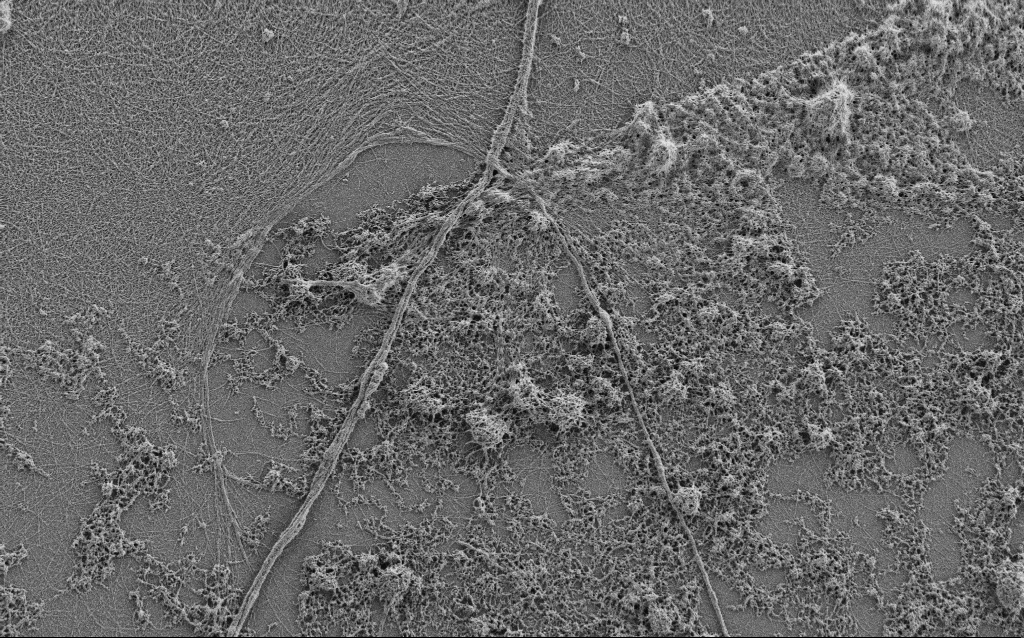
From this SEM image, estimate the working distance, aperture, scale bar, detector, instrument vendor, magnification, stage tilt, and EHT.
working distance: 4 mm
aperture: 30 µm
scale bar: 2000 nm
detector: SE2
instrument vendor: Zeiss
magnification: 7.5 K X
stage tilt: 0°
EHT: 0.9 kV